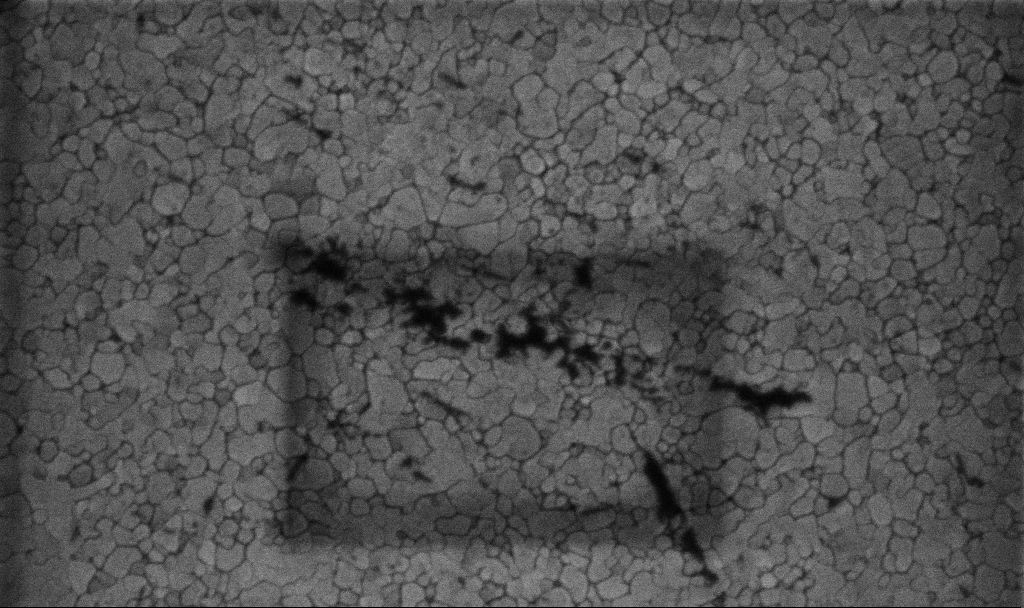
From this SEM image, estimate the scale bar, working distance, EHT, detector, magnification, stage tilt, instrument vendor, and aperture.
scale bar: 200 nm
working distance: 3.1 mm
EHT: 5 kV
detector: InLens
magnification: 100.15 K X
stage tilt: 0°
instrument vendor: Zeiss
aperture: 30 µm